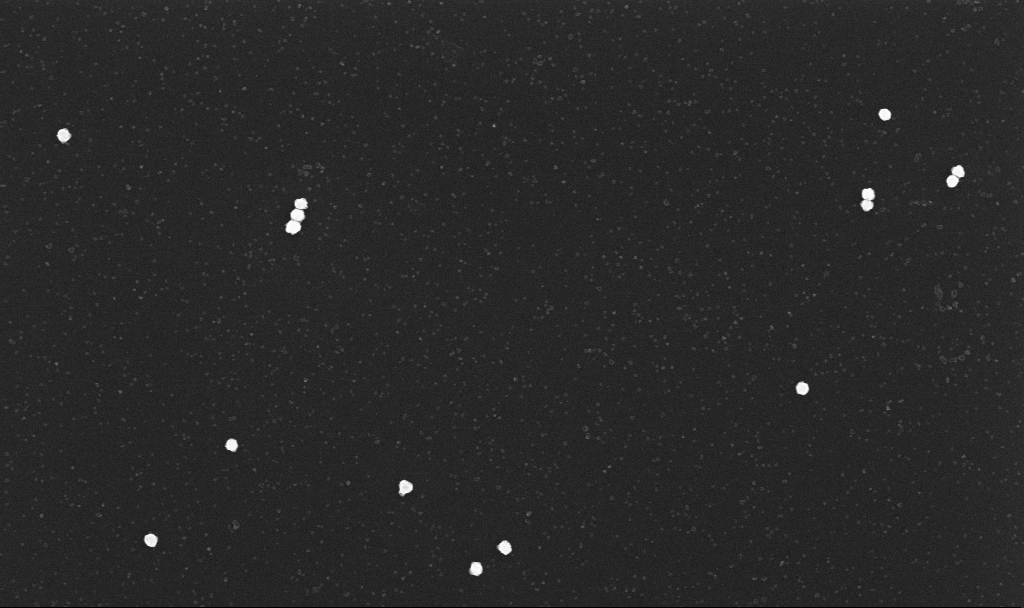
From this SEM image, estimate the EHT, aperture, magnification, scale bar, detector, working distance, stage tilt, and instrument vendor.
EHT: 10 kV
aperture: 30 µm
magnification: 70 K X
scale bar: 1000 nm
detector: InLens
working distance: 3.4 mm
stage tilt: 0°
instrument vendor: Zeiss